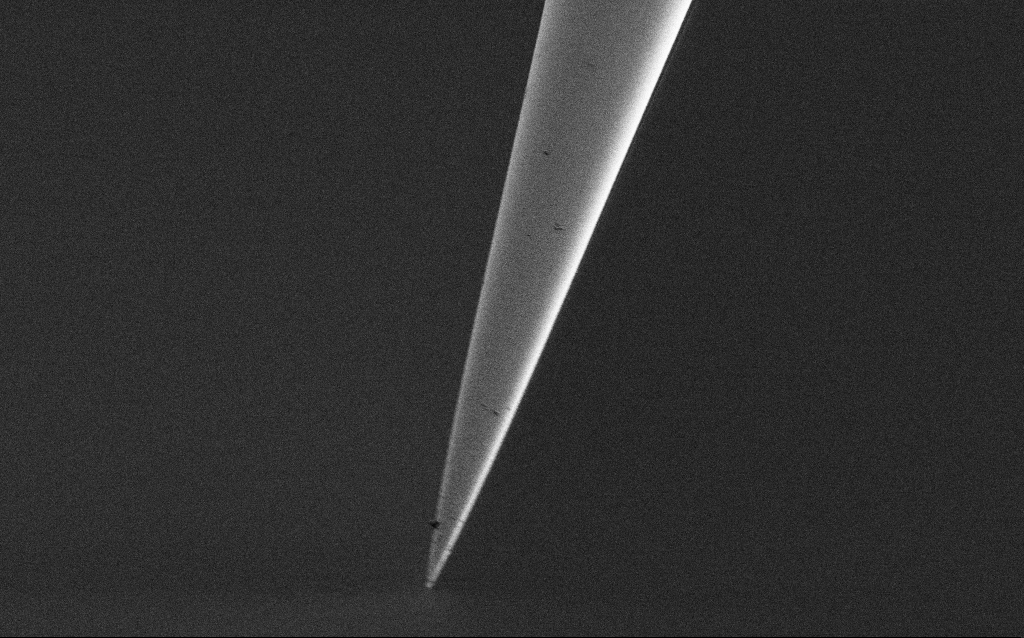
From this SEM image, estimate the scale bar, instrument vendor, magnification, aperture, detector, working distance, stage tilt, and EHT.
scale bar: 2000 nm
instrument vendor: Zeiss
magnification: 10 K X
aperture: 30 µm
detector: SE2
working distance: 6.5 mm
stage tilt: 45°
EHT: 1 kV